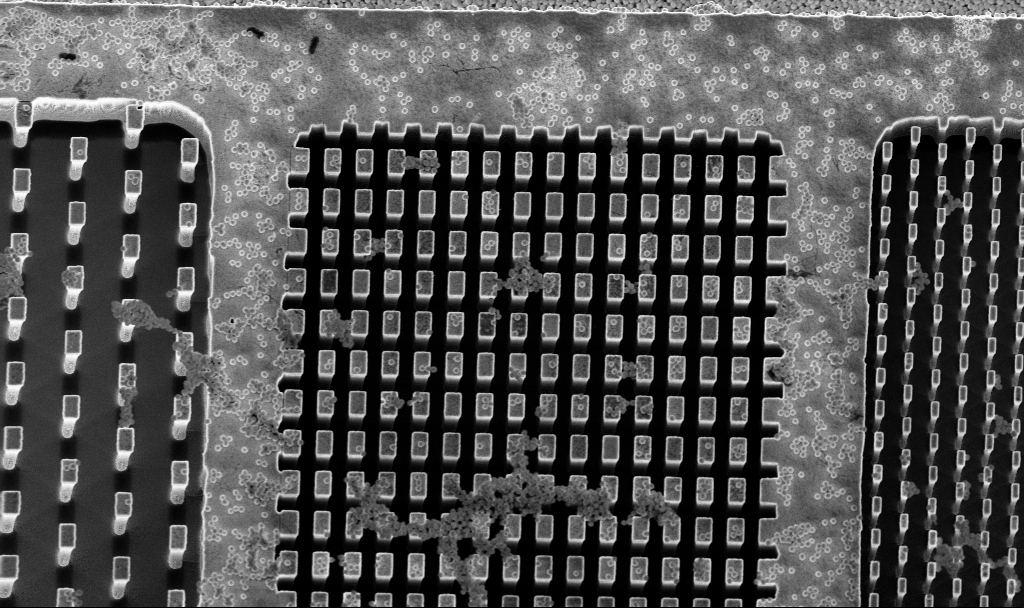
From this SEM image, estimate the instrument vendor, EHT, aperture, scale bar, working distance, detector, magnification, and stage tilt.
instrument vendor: Zeiss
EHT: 5 kV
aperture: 30 µm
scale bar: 10000 nm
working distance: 3.3 mm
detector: InLens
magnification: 3.71 K X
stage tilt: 8°